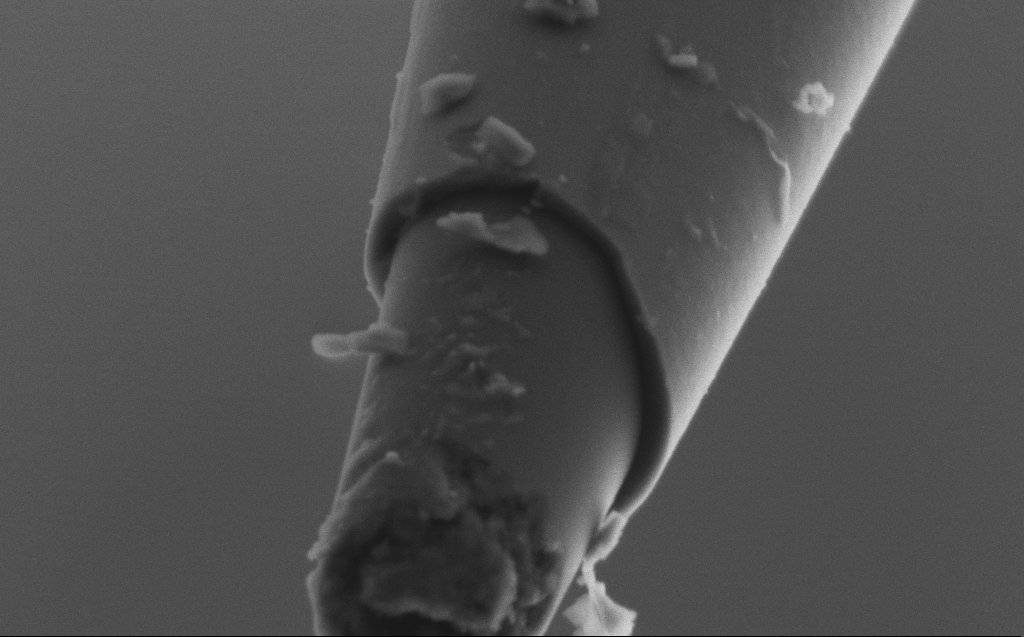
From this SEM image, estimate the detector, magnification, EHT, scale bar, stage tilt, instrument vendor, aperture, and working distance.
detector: SE2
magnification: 100 K X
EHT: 2 kV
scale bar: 200 nm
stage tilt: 45°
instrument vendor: Zeiss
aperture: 30 µm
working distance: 5 mm